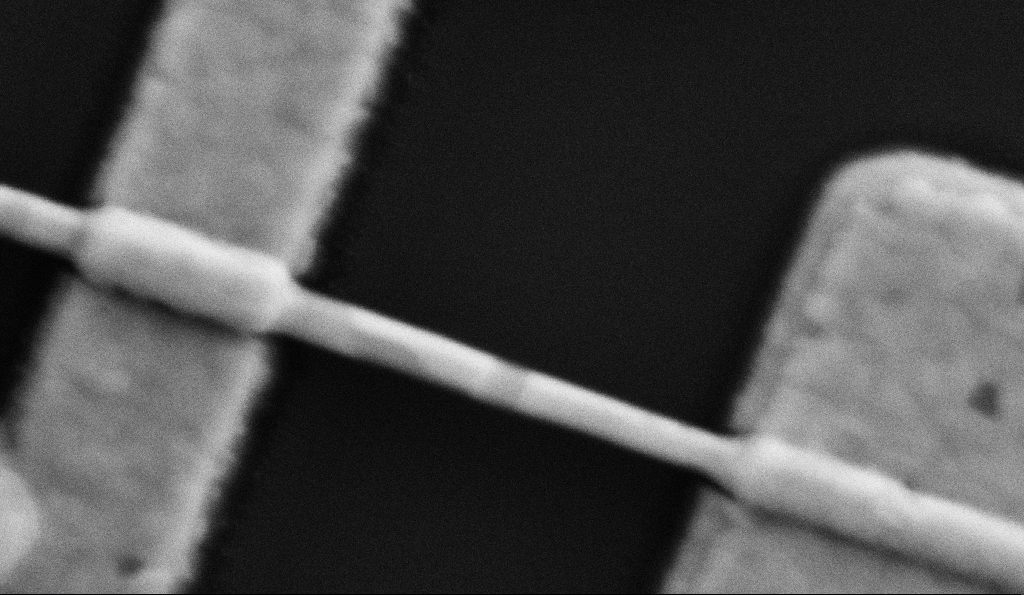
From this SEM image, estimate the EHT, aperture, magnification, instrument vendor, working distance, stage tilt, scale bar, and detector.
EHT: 5 kV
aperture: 30 µm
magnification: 200 K X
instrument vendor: Zeiss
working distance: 8.5 mm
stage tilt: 0°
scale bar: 100 nm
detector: SE2